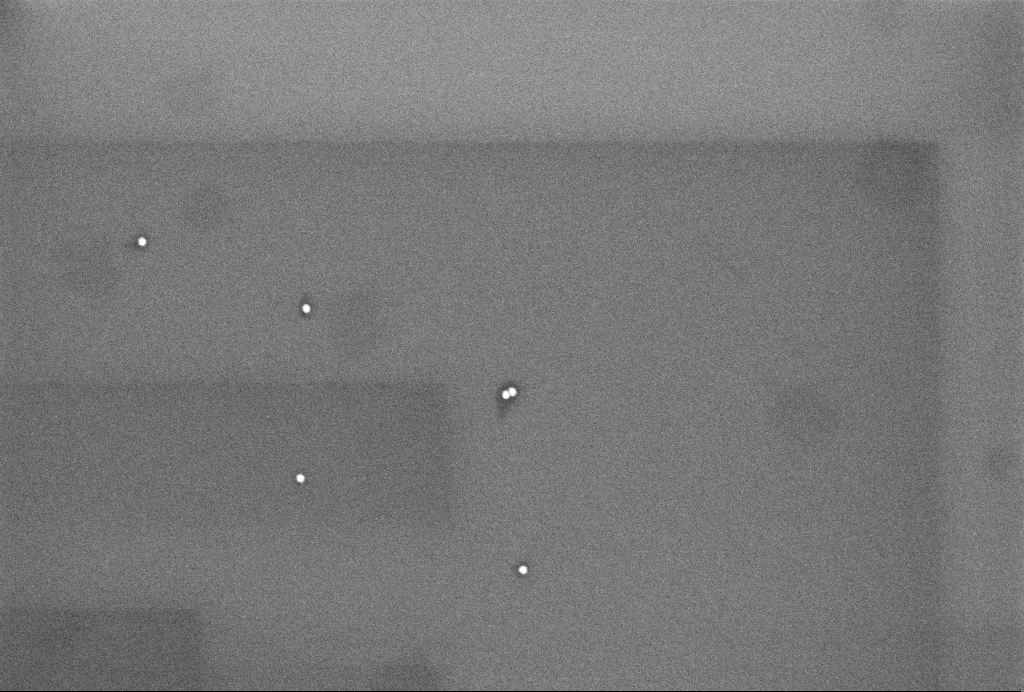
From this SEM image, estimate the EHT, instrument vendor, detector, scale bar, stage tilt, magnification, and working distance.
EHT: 3 kV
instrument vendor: Zeiss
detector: InLens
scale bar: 100 nm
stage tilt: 0°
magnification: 108.48 K X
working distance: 3.2 mm